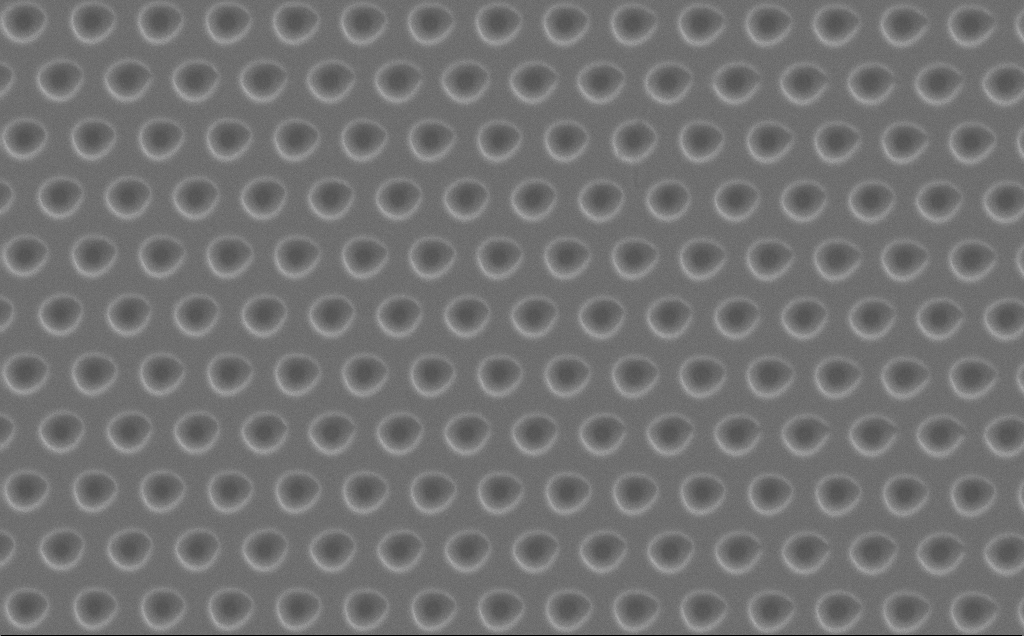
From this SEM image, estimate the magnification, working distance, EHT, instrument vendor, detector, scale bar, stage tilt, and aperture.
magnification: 6.14 K X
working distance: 10 mm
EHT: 5 kV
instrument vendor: Zeiss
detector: SE2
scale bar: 10000 nm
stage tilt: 0°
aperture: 30 µm